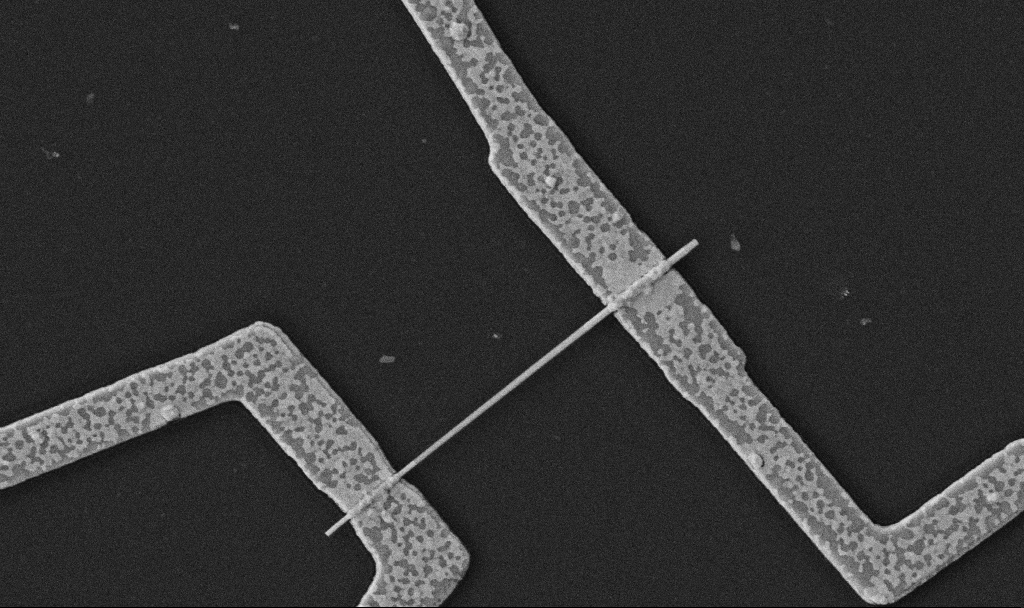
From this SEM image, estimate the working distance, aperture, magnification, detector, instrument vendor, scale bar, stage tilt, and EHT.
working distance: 10.7 mm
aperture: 30 µm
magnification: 30 K X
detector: SE2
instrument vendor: Zeiss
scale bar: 1000 nm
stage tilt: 0°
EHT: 5 kV